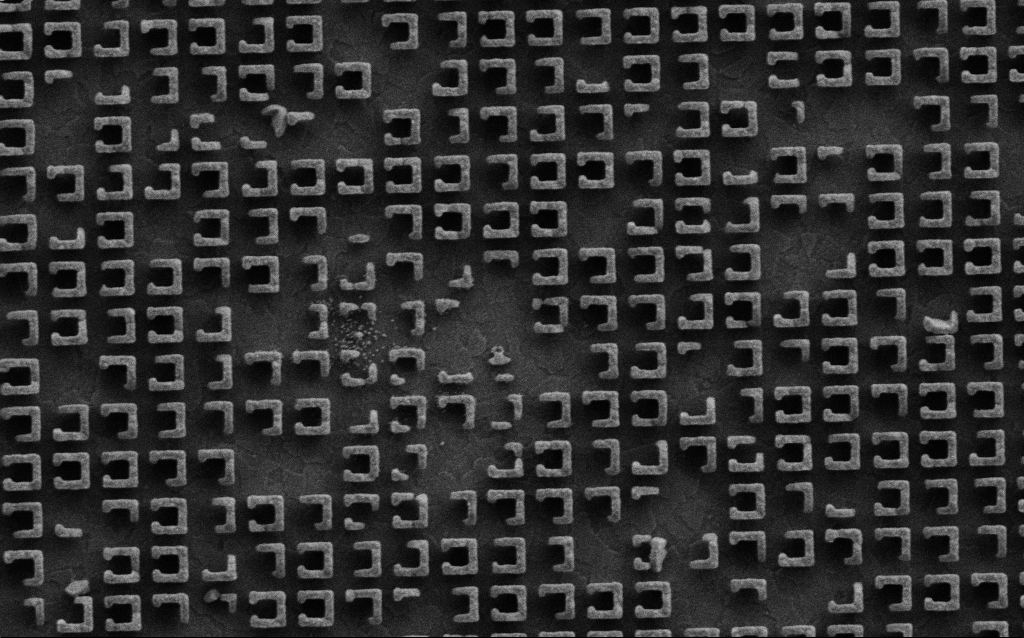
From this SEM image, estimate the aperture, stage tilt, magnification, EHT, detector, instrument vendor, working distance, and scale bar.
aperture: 30 µm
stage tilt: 0°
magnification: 38.35 K X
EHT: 3 kV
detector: SE2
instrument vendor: Zeiss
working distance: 6.6 mm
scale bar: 1000 nm